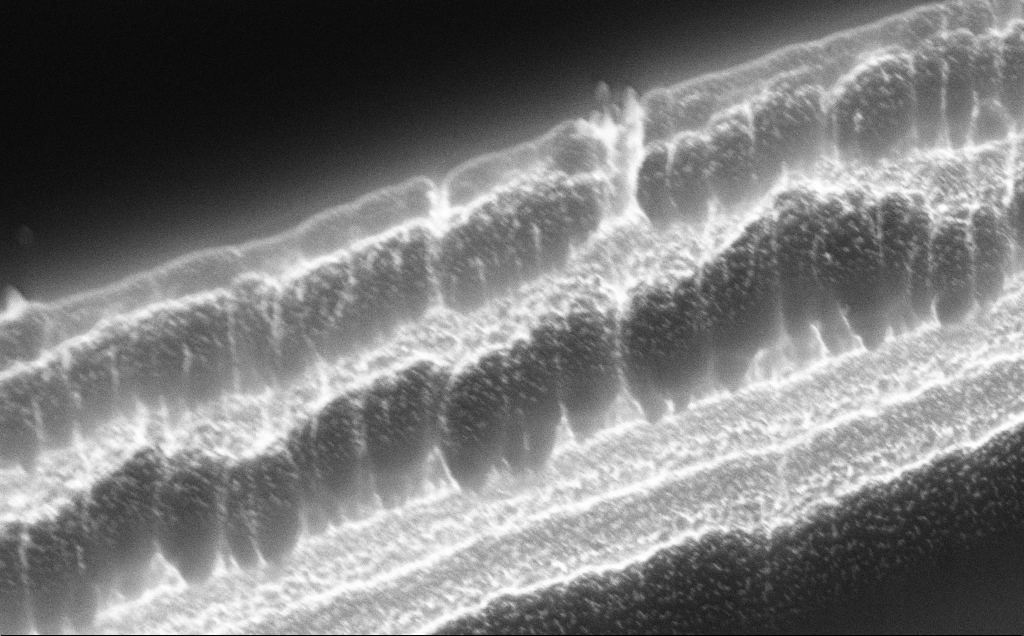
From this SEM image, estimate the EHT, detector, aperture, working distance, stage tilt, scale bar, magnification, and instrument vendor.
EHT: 10 kV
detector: InLens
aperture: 30 µm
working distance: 11 mm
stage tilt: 50°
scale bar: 1000 nm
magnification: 48.96 K X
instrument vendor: Zeiss